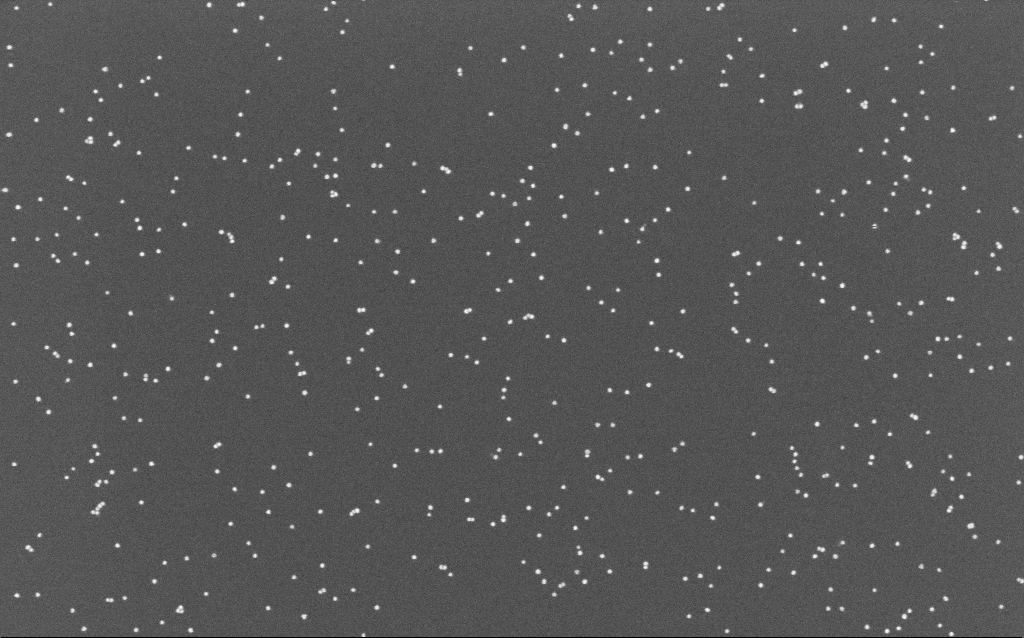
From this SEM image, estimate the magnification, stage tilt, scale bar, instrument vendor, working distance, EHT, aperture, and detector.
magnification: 100 K X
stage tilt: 0°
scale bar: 200 nm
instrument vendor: Zeiss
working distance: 6.6 mm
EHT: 10 kV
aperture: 30 µm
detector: InLens